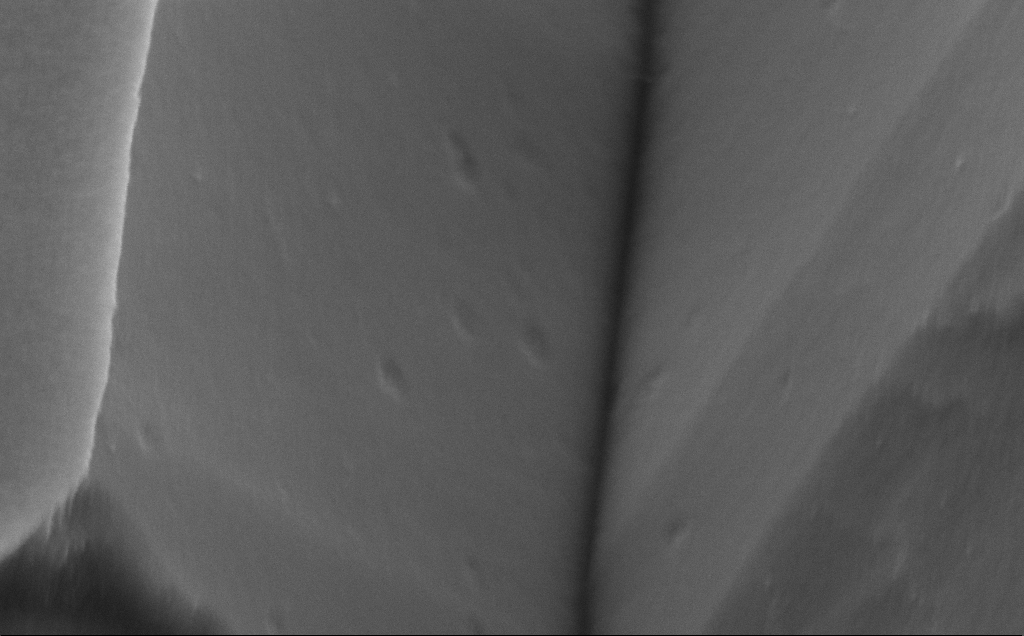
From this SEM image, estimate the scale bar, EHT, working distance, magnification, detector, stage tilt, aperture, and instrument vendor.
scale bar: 200 nm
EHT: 5 kV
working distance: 10 mm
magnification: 116.5 K X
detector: SE2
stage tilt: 50°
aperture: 30 µm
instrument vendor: Zeiss